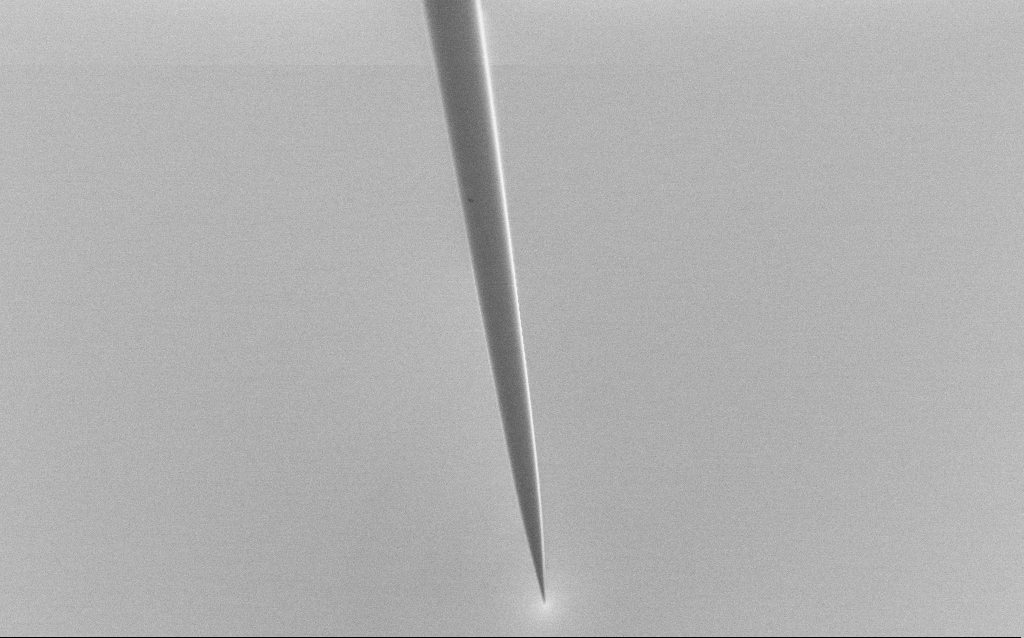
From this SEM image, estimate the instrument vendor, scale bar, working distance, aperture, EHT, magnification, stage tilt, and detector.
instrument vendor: Zeiss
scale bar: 100000 nm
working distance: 6 mm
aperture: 30 µm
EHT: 1 kV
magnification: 0.5 K X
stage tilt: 45°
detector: SE2